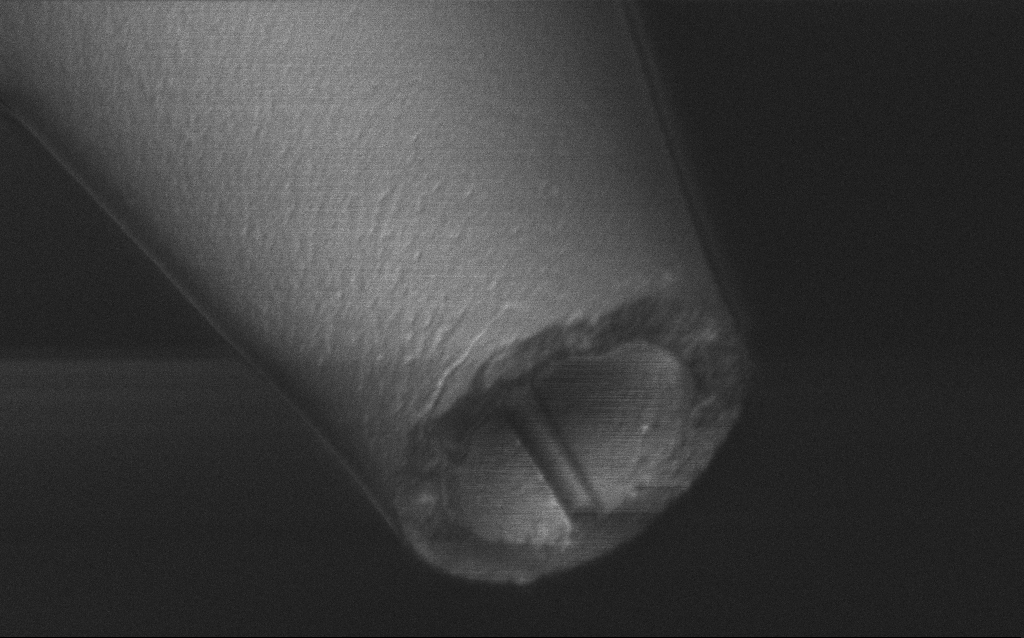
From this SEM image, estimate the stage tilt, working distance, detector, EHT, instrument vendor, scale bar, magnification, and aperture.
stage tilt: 45°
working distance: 7 mm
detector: InLens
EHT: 1 kV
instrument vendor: Zeiss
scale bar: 1000 nm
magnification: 50 K X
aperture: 30 µm